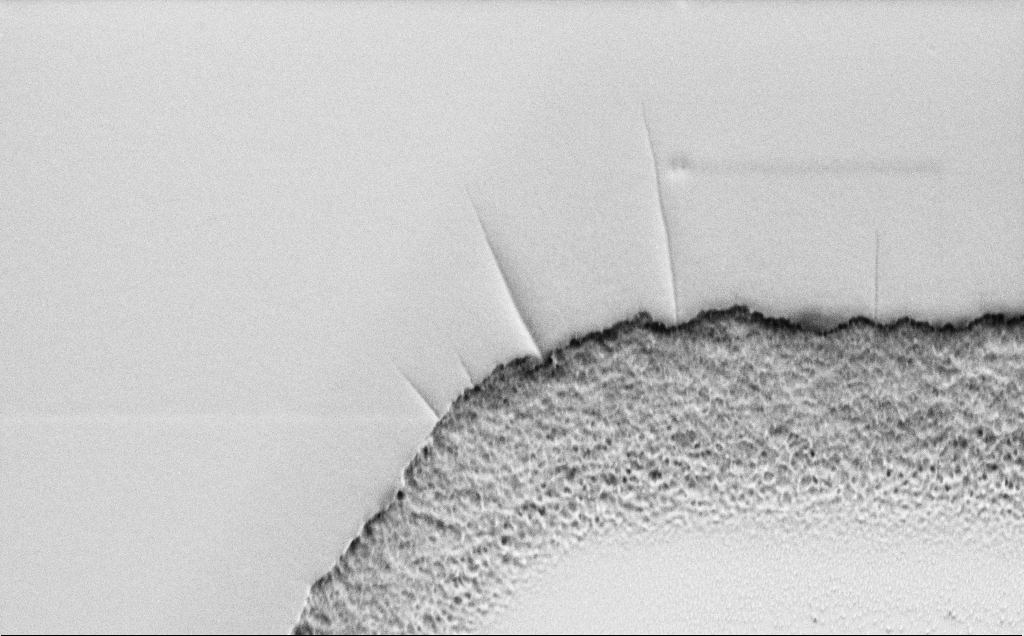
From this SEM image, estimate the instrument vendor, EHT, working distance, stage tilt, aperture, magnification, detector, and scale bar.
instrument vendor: Zeiss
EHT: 1.5 kV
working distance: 6 mm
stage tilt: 45°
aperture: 30 µm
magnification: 24.86 K X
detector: SE2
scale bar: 2000 nm